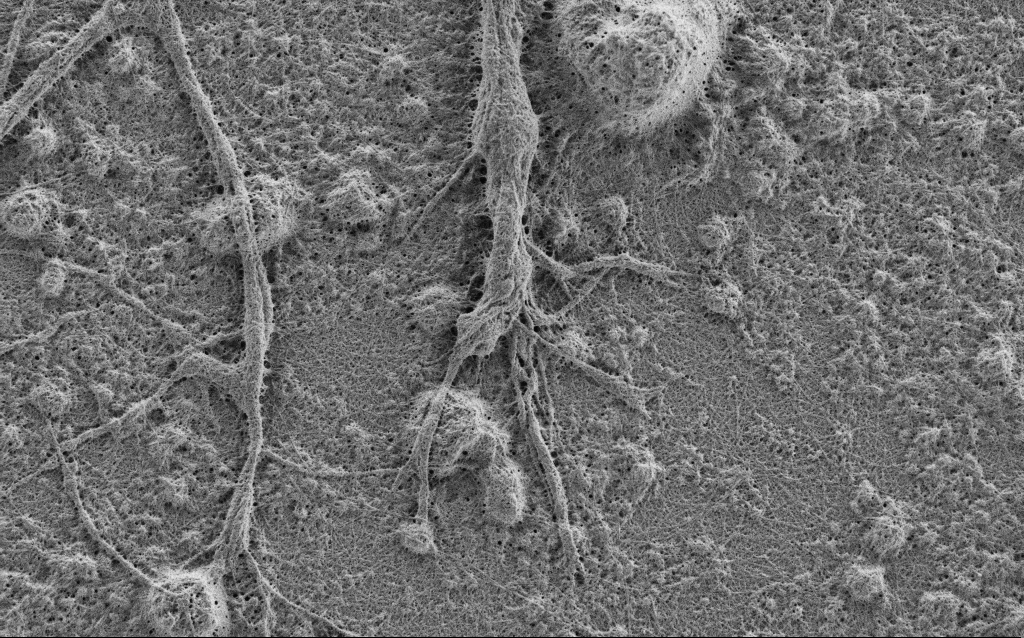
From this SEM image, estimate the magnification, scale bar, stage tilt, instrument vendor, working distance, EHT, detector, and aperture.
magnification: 10 K X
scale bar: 2000 nm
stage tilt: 0°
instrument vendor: Zeiss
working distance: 4 mm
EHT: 0.9 kV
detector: SE2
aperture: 30 µm